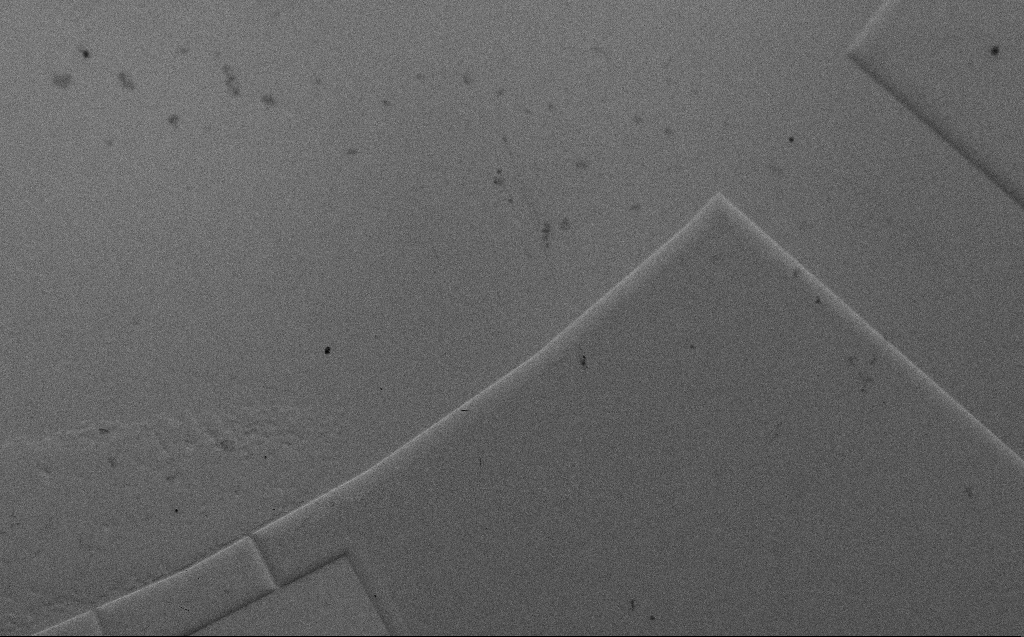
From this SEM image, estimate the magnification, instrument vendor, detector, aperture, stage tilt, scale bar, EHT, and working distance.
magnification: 0.179 K X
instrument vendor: Zeiss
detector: SE2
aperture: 30 µm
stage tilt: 45°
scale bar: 200000 nm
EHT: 5 kV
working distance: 7 mm